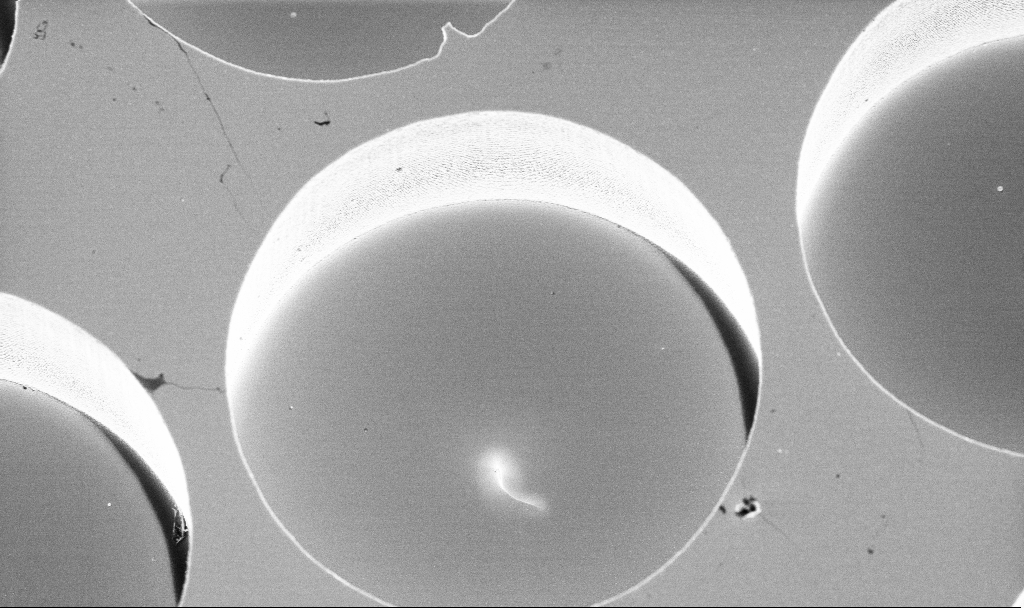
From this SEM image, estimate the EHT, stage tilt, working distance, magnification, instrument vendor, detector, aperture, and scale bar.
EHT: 4 kV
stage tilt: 15°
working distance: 4.7 mm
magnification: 6.63 K X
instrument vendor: Zeiss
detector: InLens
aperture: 30 µm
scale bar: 10000 nm